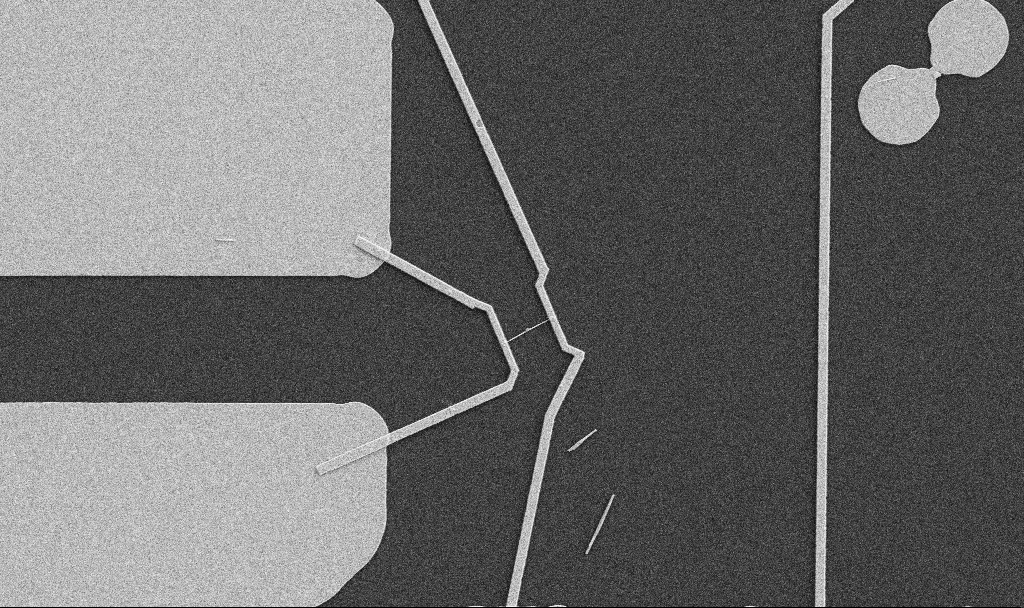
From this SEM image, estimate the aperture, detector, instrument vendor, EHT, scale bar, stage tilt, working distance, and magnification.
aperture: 30 µm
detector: SE2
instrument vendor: Zeiss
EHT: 5 kV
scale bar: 10000 nm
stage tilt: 0°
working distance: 10.7 mm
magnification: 5 K X